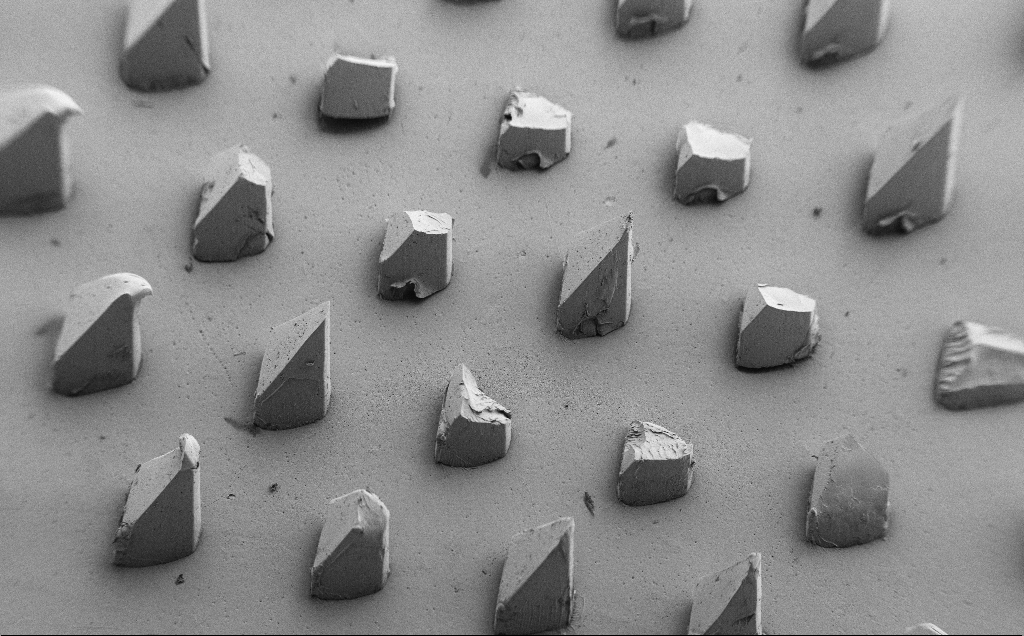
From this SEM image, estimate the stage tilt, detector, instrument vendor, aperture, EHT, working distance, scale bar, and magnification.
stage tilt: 39°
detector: SE2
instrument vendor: Zeiss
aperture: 30 µm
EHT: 5 kV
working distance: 8 mm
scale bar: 1e+06 nm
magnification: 0.071 K X